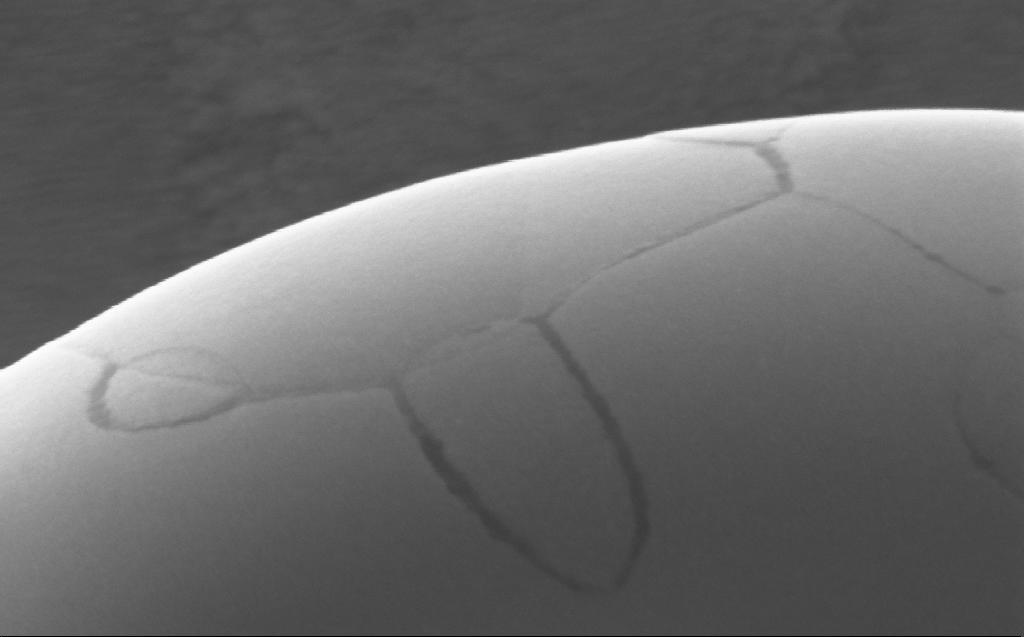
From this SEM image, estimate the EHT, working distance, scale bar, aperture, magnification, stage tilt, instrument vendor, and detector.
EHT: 5 kV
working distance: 2 mm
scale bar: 200 nm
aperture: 30 µm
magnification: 272.09 K X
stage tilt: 0°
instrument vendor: Zeiss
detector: InLens